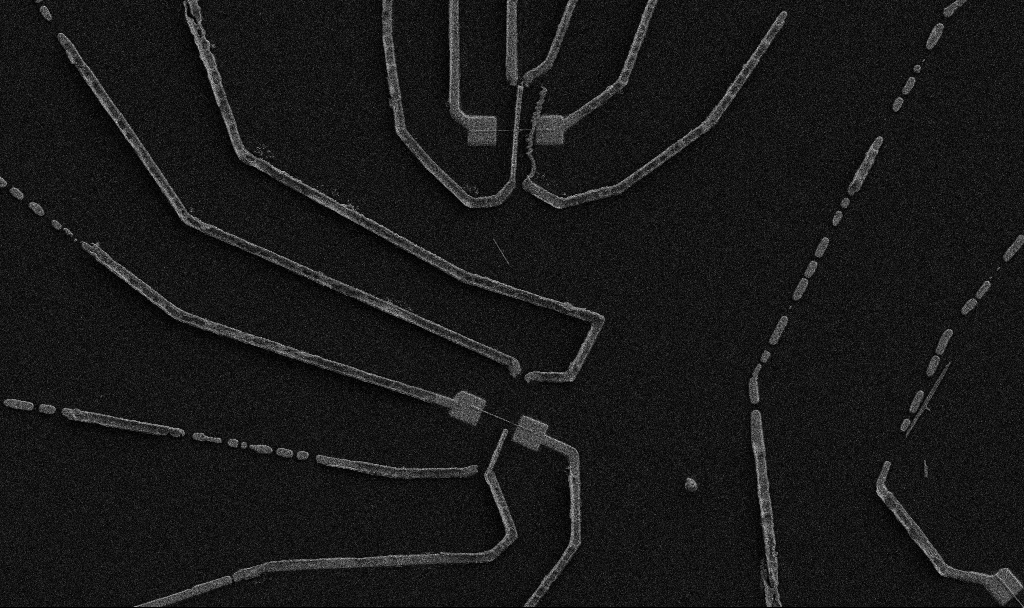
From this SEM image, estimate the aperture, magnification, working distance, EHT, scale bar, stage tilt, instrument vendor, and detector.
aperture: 30 µm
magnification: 5 K X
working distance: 10.7 mm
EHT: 5 kV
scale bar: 10000 nm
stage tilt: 0°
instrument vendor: Zeiss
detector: SE2